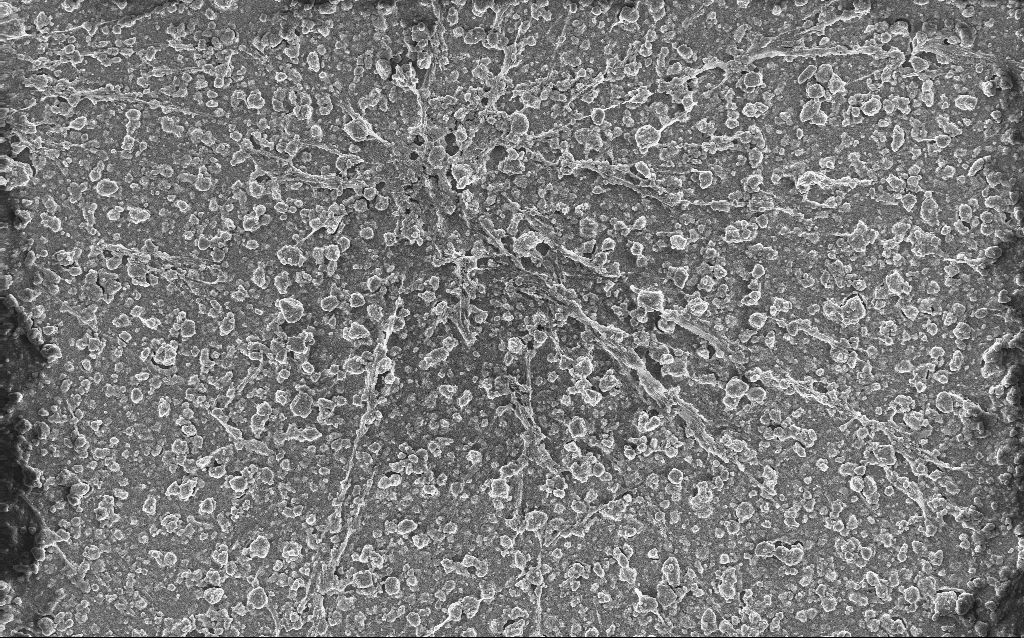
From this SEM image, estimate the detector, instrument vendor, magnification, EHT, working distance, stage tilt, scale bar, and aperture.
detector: InLens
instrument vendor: Zeiss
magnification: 0.792 K X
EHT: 10 kV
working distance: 2.8 mm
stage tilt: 0°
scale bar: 20000 nm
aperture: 30 µm